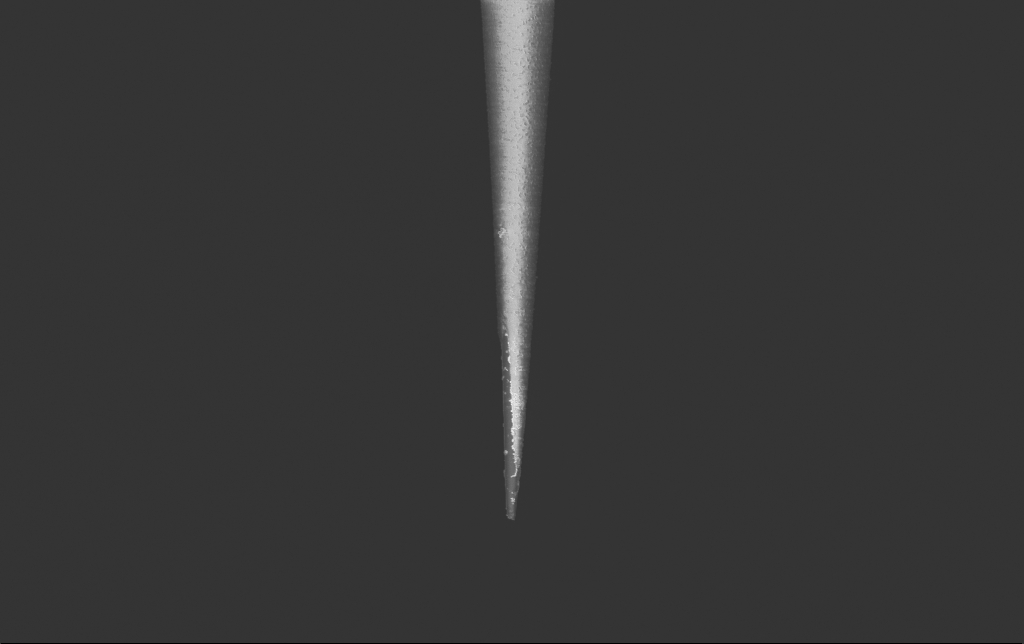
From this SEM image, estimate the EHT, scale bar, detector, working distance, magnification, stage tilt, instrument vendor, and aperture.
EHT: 2 kV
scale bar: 10000 nm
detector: InLens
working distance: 5.9 mm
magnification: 5 K X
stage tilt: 0°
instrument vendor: Zeiss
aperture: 30 µm